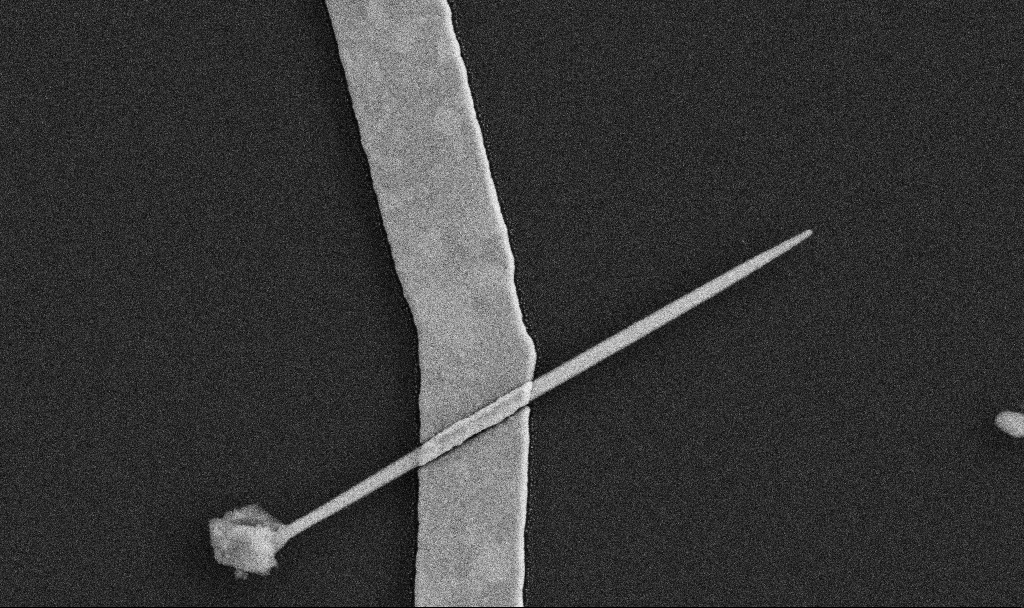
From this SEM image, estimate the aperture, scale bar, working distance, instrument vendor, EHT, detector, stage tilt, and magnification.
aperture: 30 µm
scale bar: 1000 nm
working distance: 7.6 mm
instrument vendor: Zeiss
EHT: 5 kV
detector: SE2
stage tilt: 0°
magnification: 45.95 K X